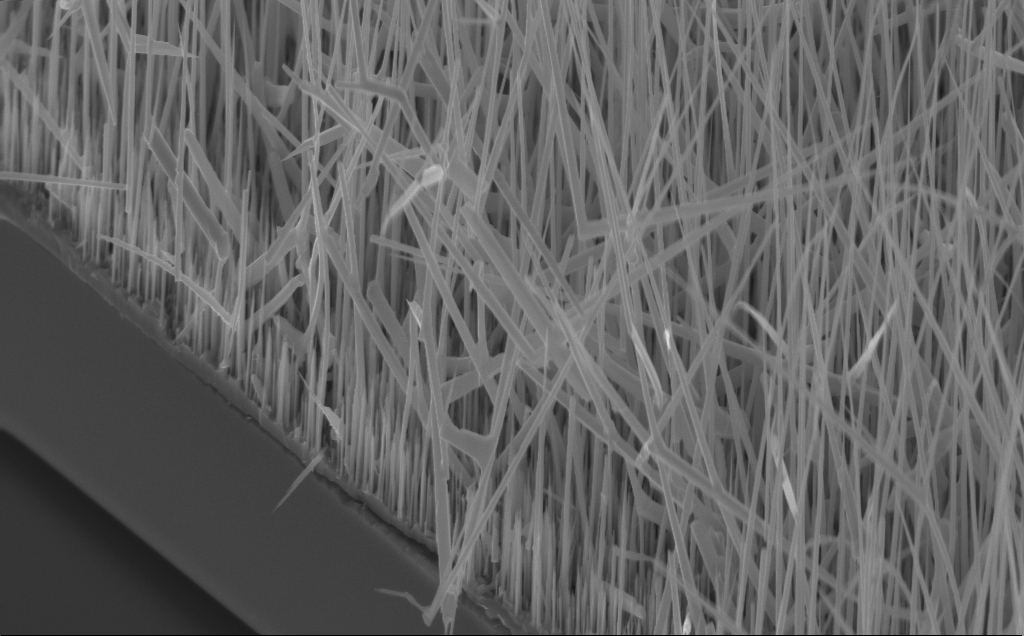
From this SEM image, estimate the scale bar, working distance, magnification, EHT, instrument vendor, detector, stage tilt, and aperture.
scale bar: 2000 nm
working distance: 5 mm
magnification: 16.76 K X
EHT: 10 kV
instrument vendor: Zeiss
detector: InLens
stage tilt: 45°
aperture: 30 µm